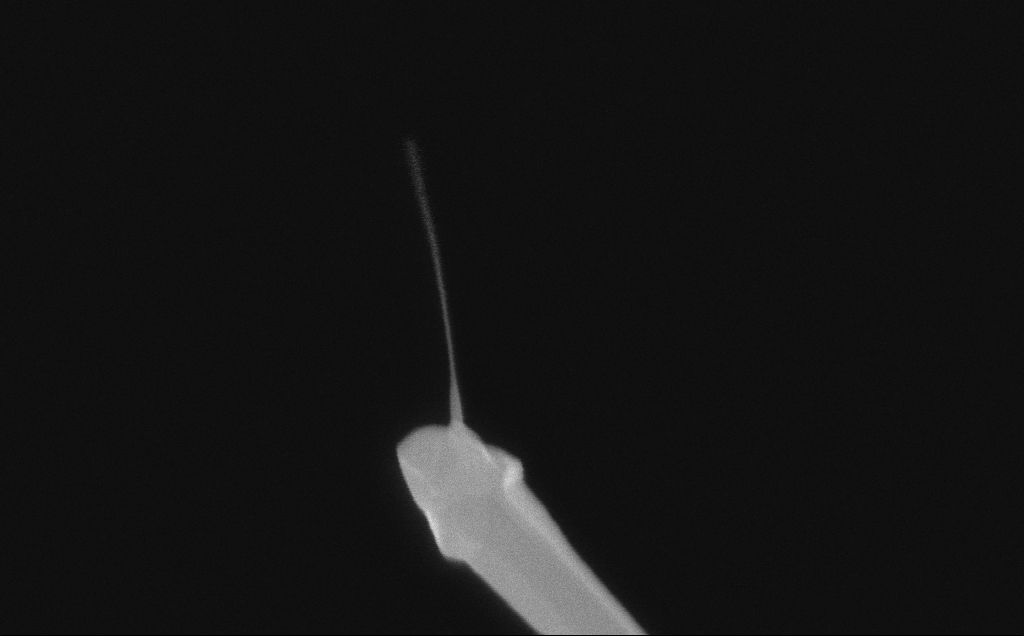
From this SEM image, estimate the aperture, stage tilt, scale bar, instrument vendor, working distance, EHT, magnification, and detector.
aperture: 30 µm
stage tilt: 0°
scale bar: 200 nm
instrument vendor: Zeiss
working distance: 6 mm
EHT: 10 kV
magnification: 189.64 K X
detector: InLens